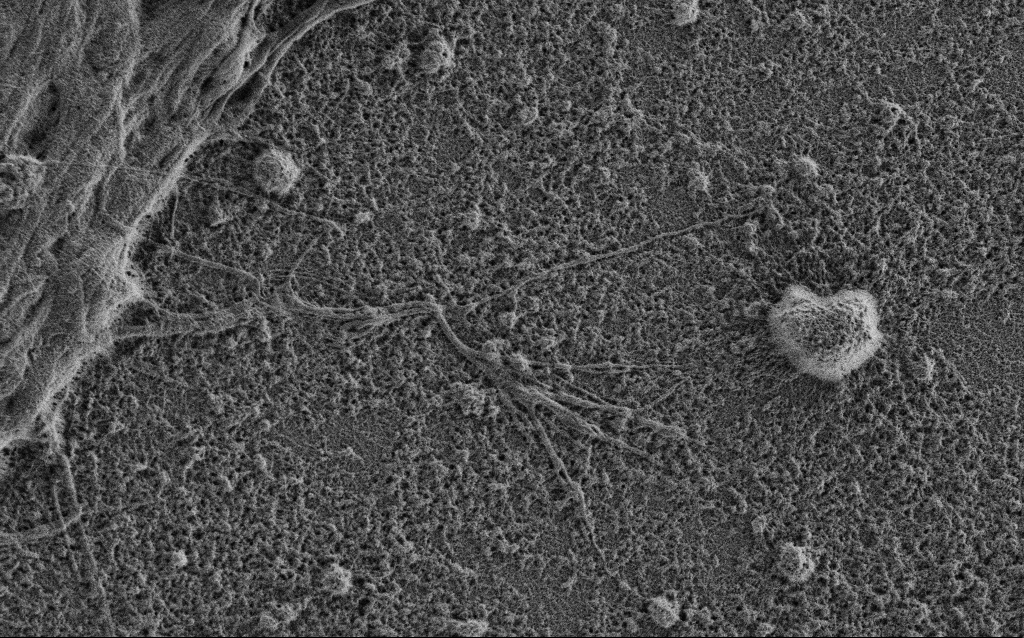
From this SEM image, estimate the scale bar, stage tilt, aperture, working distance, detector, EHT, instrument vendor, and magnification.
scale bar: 2000 nm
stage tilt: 0°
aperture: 30 µm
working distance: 3.4 mm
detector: SE2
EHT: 0.9 kV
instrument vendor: Zeiss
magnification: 10 K X